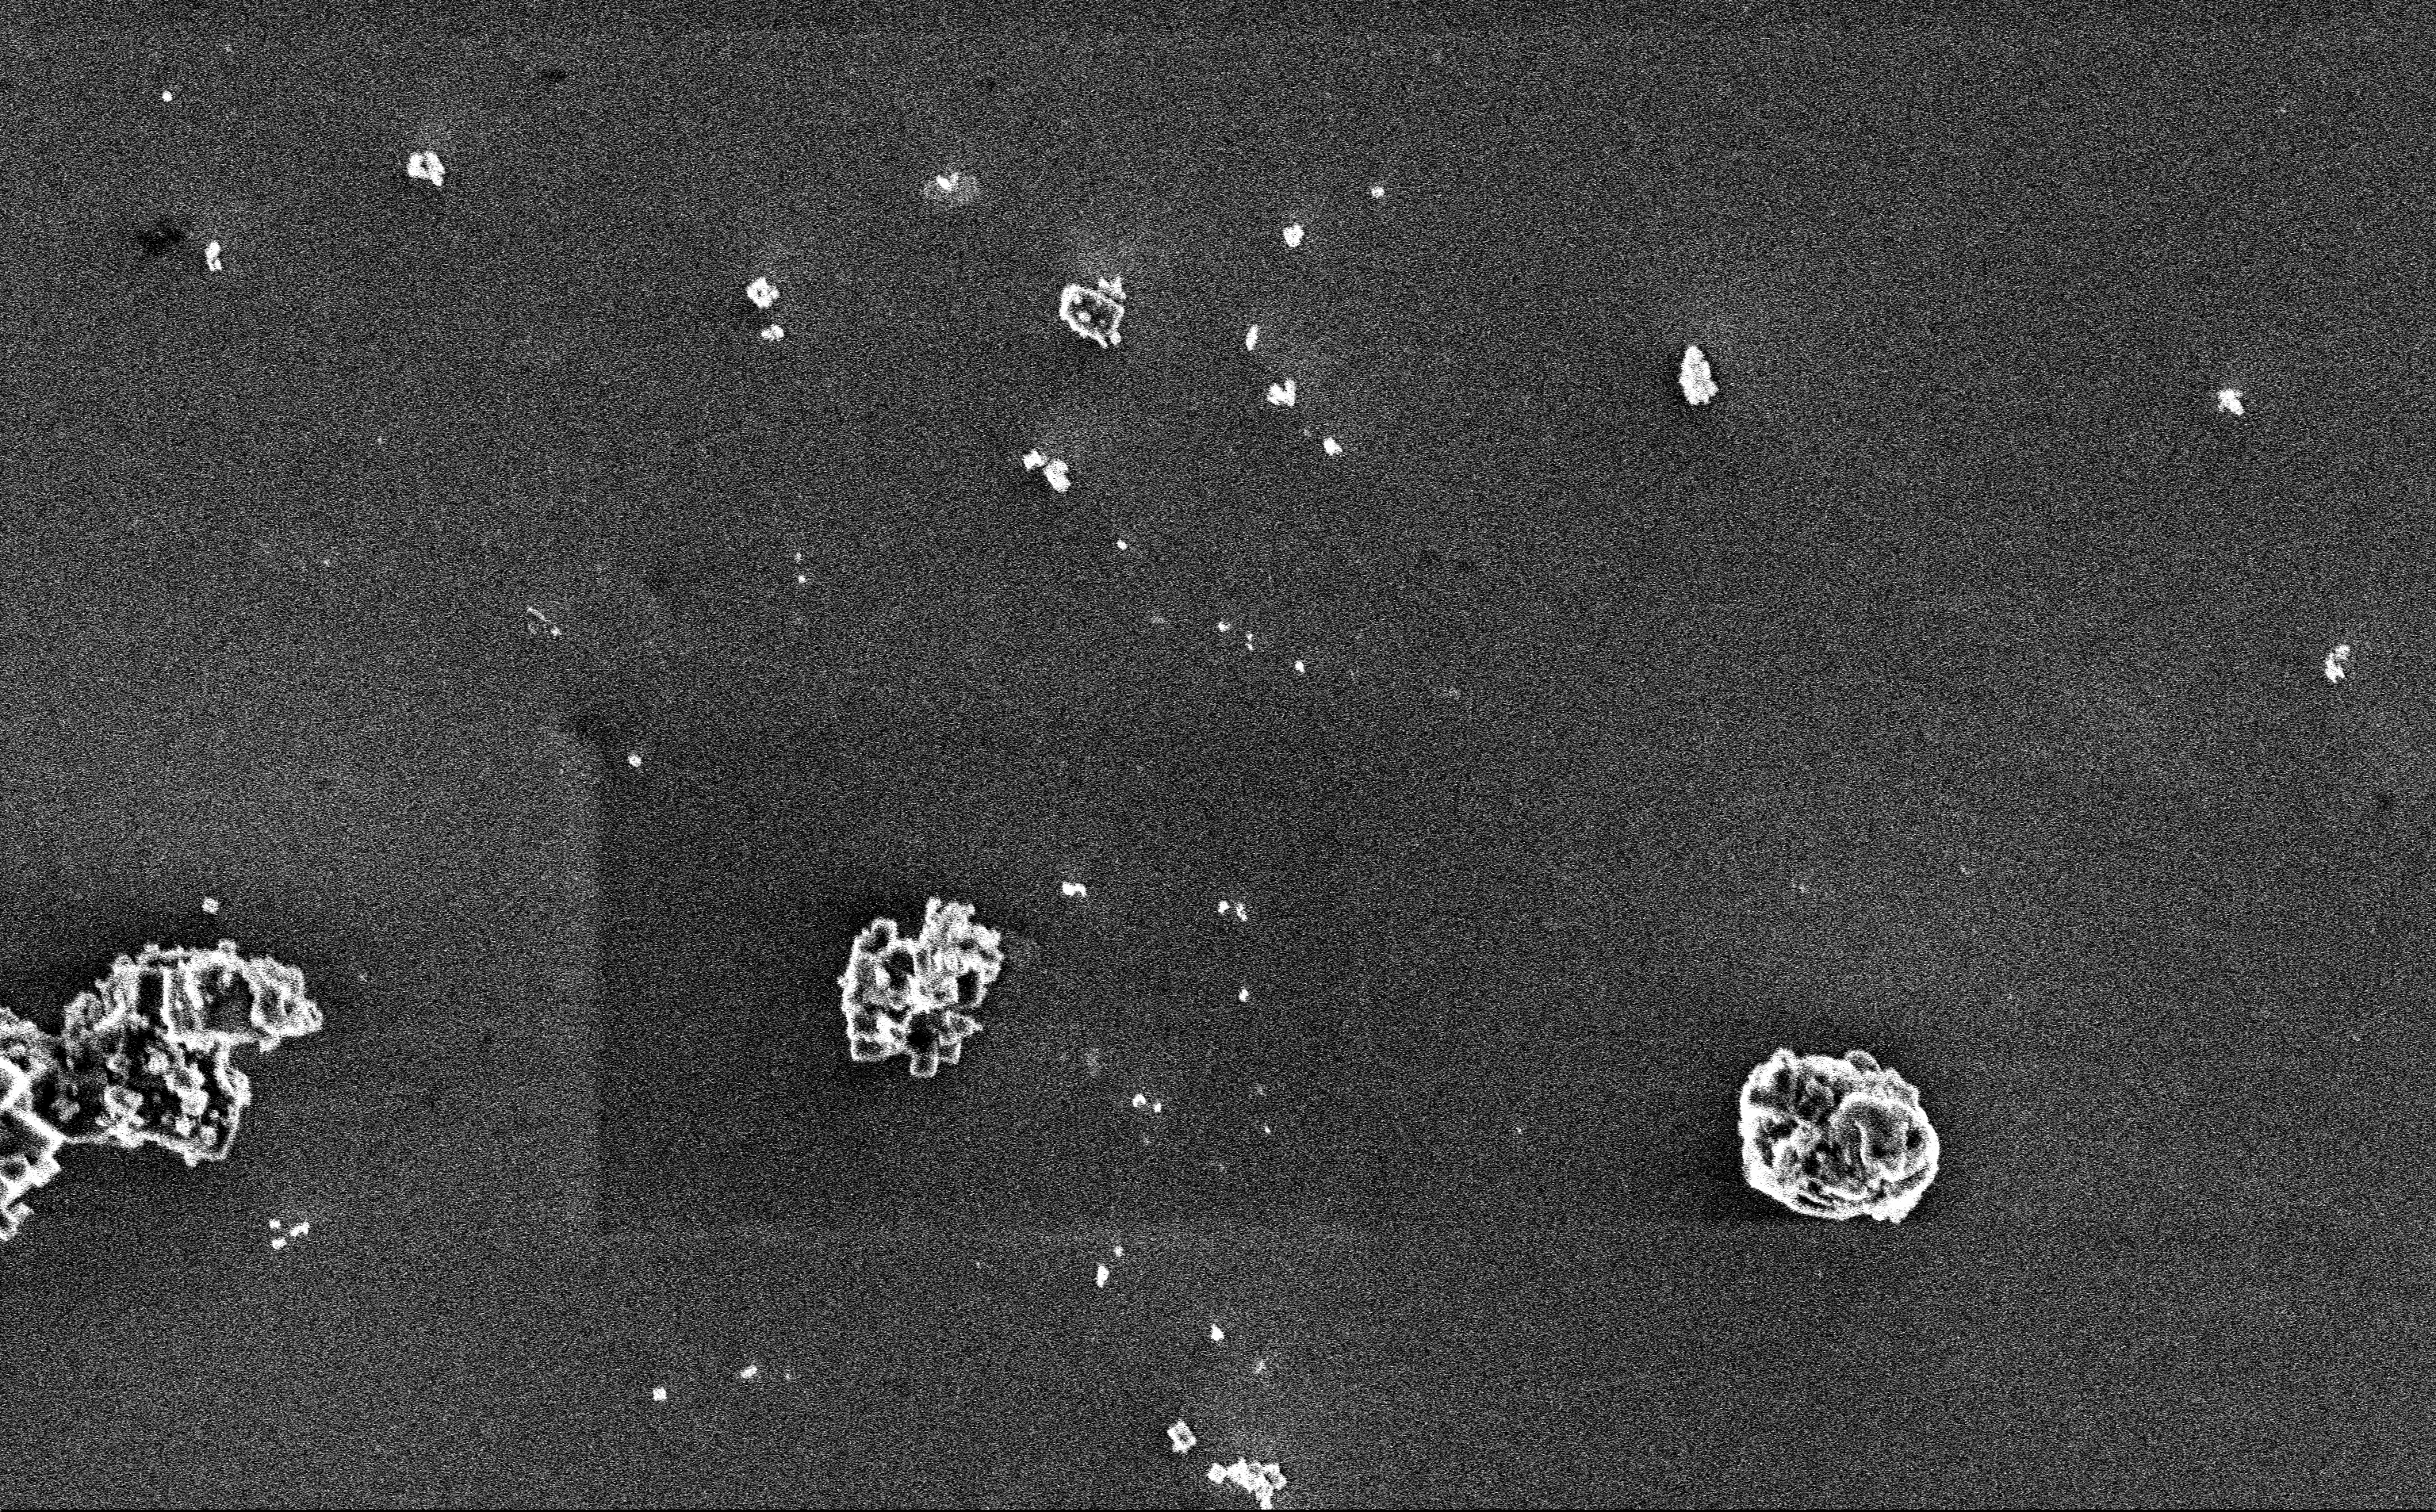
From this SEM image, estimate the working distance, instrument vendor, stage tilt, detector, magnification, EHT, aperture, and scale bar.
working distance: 3 mm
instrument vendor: Zeiss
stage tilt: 0°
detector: InLens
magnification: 12.85 K X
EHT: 3 kV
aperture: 30 µm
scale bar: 2000 nm